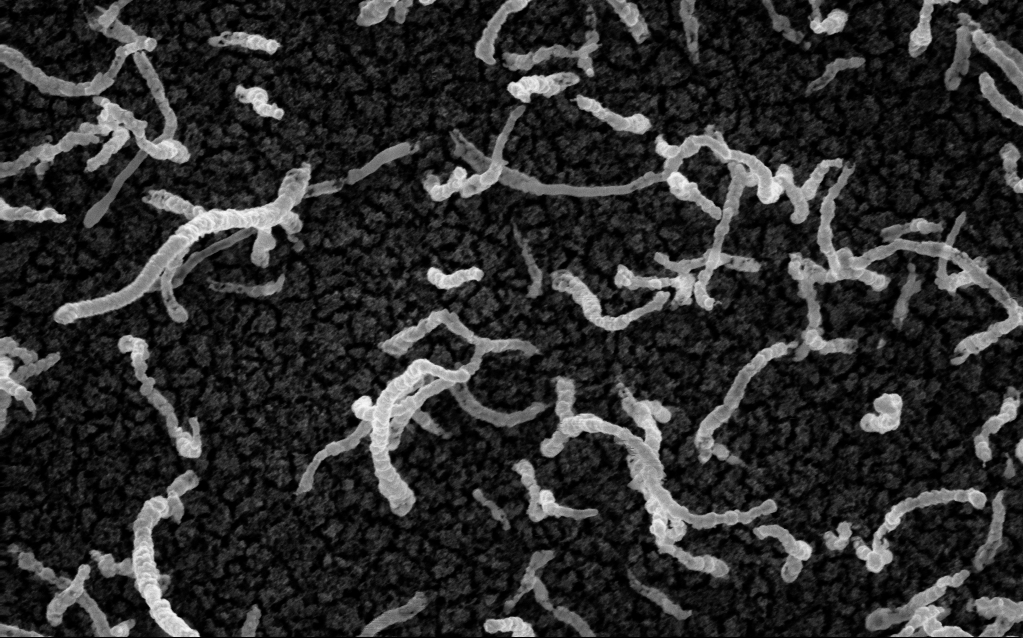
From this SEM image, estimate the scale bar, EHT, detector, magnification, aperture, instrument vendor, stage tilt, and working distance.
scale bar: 200 nm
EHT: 5 kV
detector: InLens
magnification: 100 K X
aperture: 30 µm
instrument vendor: Zeiss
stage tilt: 0°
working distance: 2.1 mm